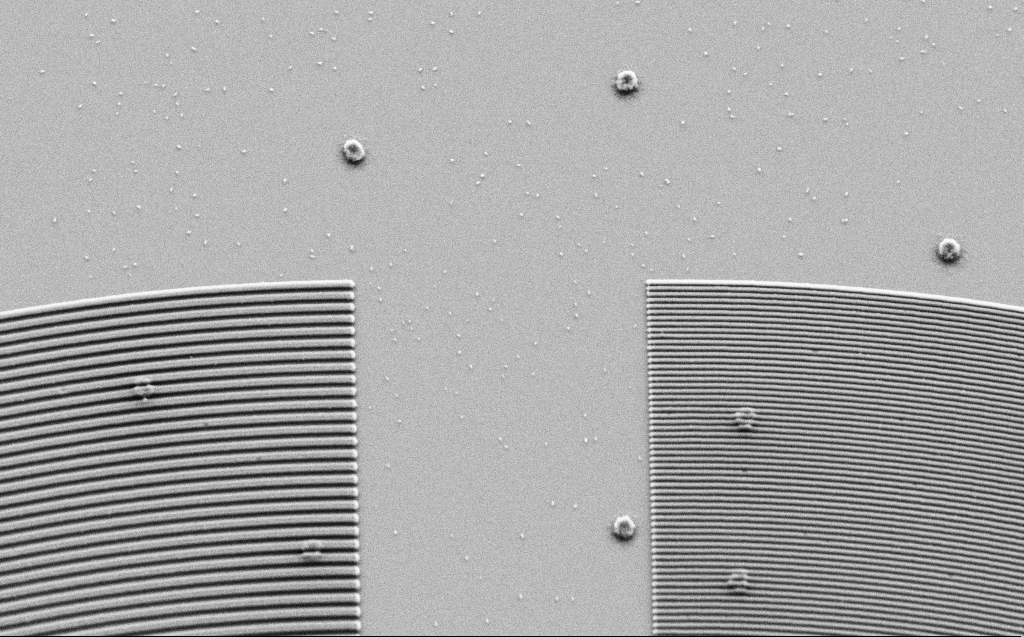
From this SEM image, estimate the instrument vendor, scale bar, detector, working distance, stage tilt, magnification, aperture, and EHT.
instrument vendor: Zeiss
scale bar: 2000 nm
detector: SE2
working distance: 6 mm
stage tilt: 30°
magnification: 10.85 K X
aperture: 30 µm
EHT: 3 kV